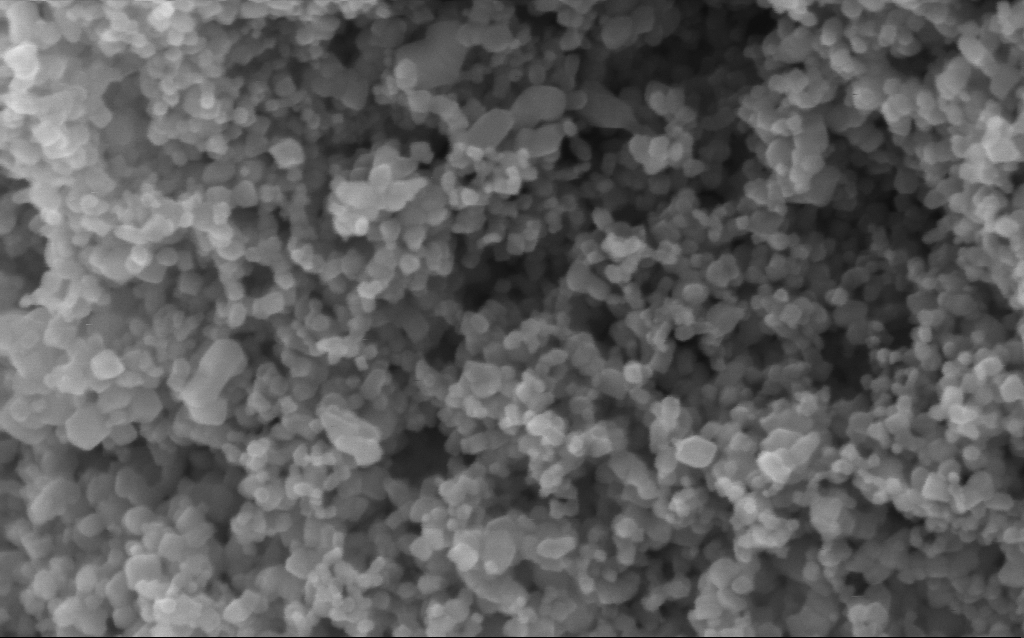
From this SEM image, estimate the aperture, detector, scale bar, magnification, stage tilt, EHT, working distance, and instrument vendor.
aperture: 30 µm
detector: InLens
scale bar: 200 nm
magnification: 294.6 K X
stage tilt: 0°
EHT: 5 kV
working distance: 4.5 mm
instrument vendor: Zeiss